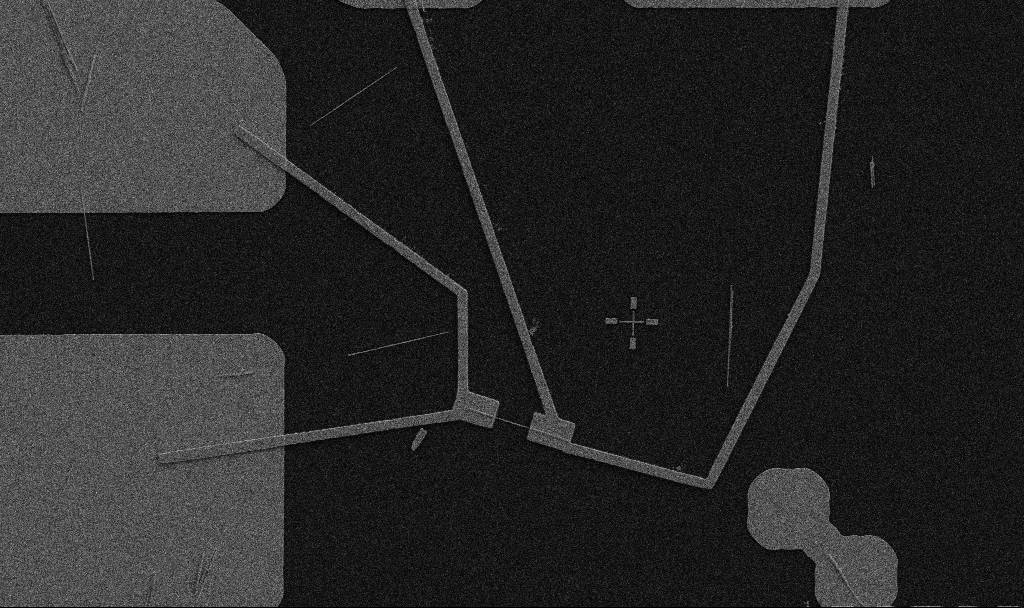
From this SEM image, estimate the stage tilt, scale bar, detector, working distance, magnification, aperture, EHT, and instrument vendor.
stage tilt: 0°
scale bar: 10000 nm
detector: SE2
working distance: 10.7 mm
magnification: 5 K X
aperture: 30 µm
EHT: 5 kV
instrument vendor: Zeiss